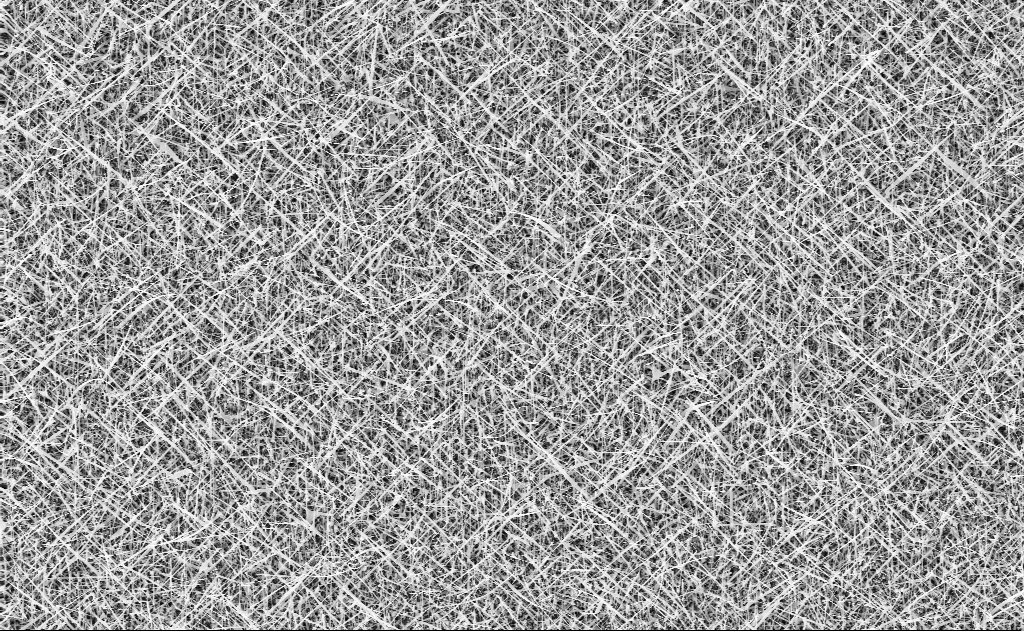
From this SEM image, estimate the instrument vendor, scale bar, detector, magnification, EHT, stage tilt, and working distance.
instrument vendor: Zeiss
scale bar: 10000 nm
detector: InLens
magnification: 5 K X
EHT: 10 kV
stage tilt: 0°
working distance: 10 mm